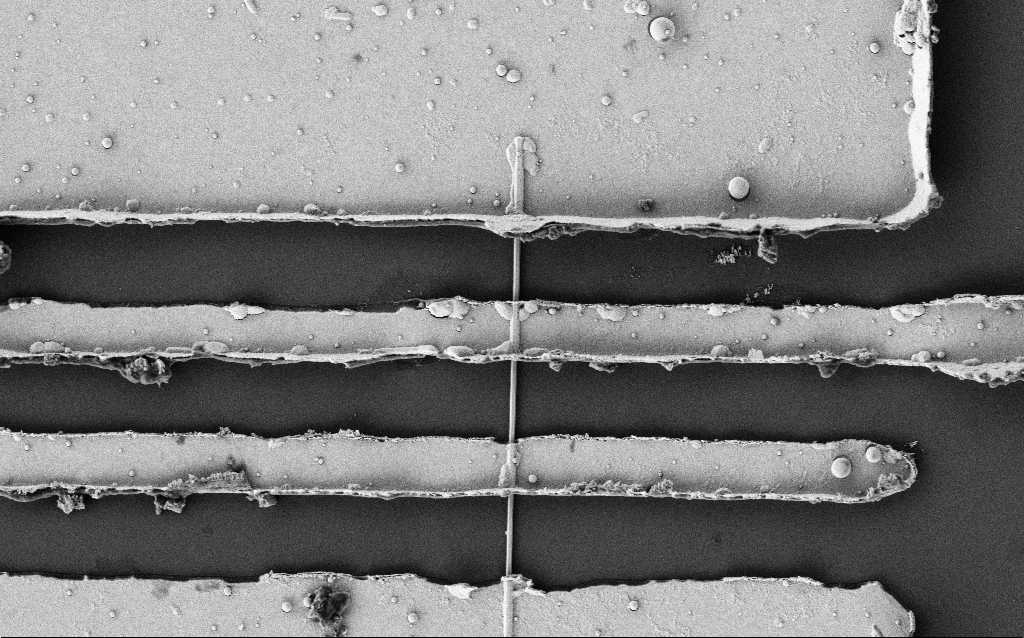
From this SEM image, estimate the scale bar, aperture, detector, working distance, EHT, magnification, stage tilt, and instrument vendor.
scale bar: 1000 nm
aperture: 30 µm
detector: SE2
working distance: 7.4 mm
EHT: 2 kV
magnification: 12.45 K X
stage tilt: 0°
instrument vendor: Zeiss